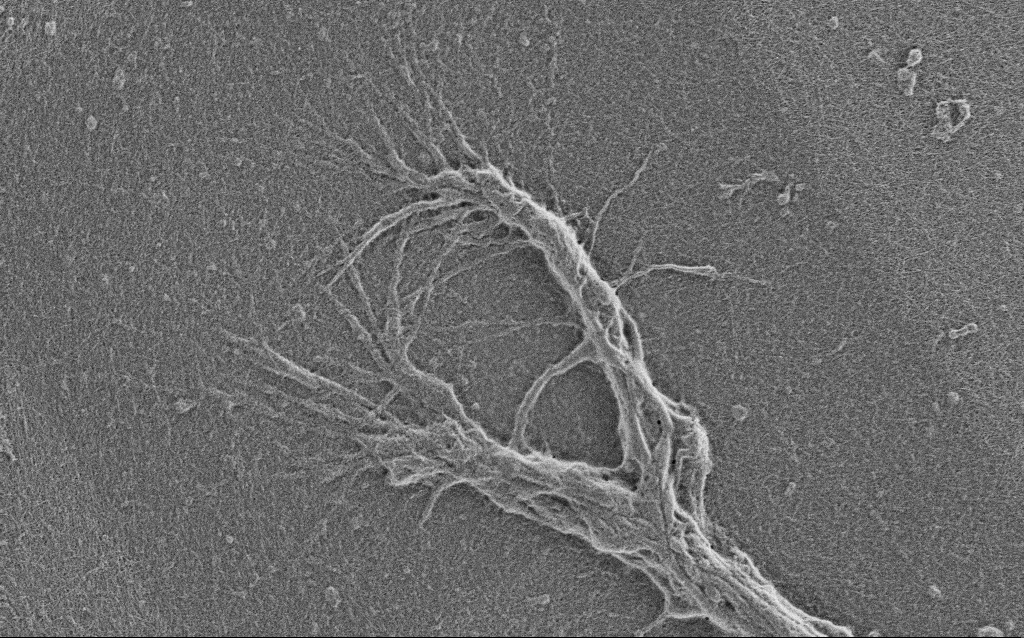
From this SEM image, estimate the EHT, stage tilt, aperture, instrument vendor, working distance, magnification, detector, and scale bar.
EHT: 1 kV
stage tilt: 0°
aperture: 30 µm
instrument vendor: Zeiss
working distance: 4 mm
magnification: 7.5 K X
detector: SE2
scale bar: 2000 nm